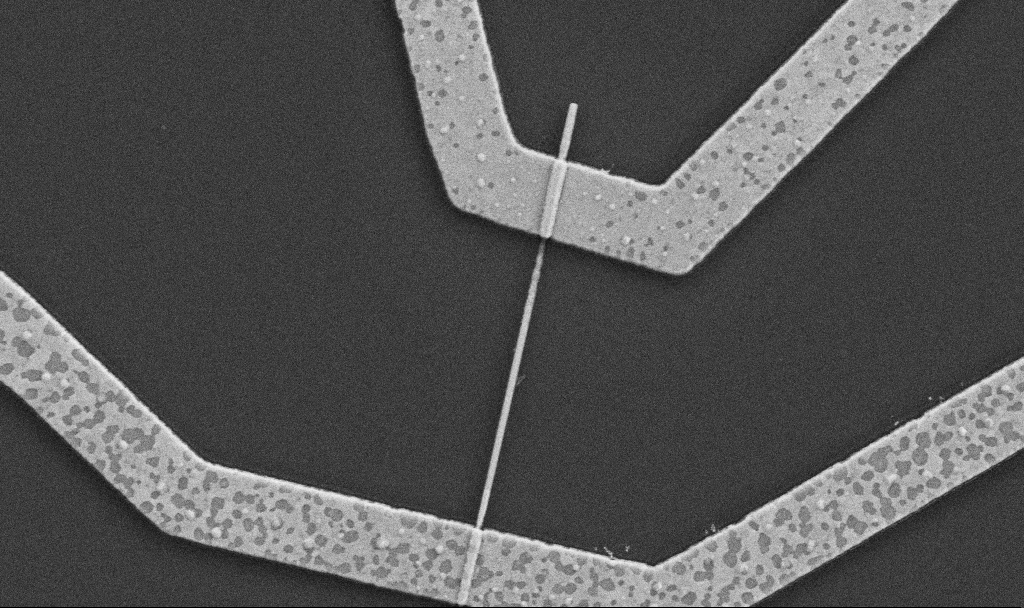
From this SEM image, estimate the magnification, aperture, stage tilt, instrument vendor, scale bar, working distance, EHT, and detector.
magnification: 30 K X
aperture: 30 µm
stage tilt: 0°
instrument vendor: Zeiss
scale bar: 1000 nm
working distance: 8.7 mm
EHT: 5 kV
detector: SE2